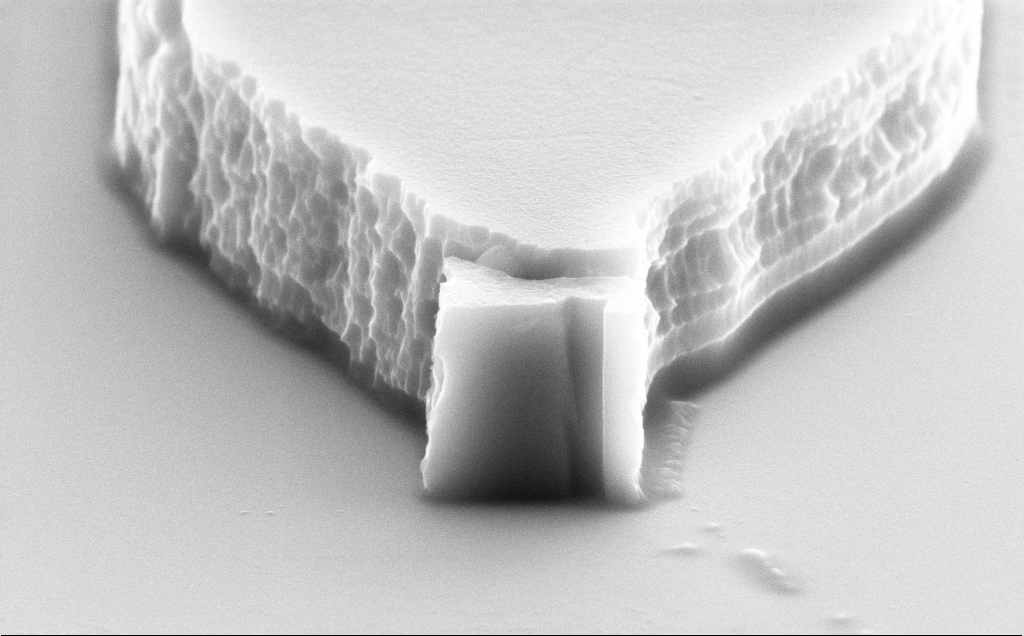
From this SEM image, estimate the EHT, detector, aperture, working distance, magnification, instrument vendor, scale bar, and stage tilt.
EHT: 10 kV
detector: SE2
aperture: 30 µm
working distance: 9 mm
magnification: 34.44 K X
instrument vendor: Zeiss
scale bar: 2000 nm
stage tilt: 70°